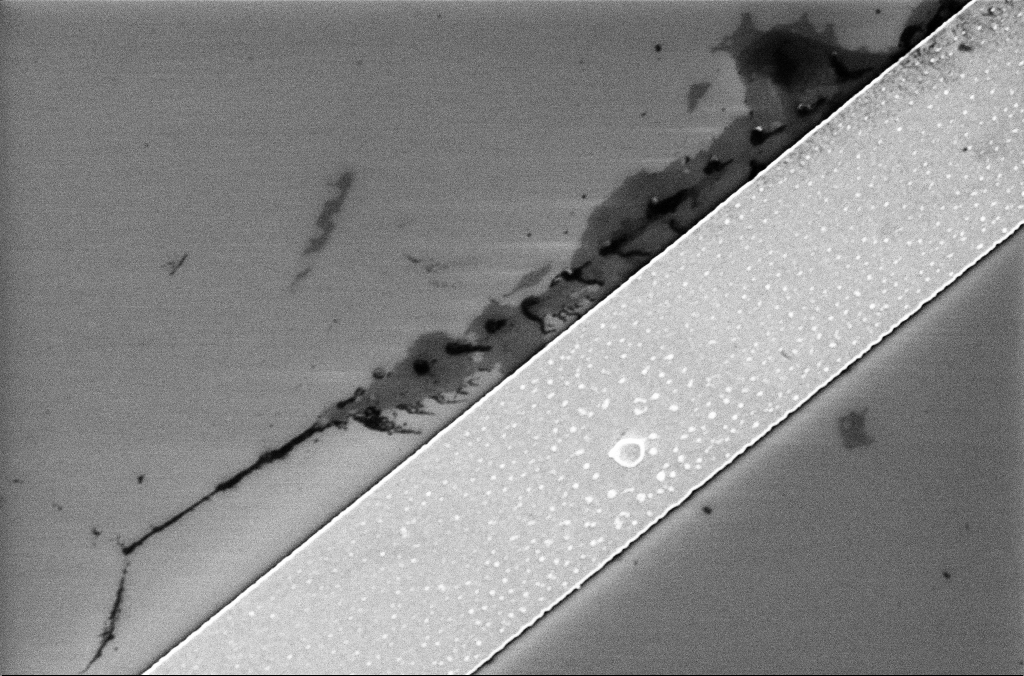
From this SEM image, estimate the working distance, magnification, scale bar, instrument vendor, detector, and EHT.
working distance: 3.4 mm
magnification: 15.13 K X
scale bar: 2000 nm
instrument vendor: Zeiss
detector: InLens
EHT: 5 kV